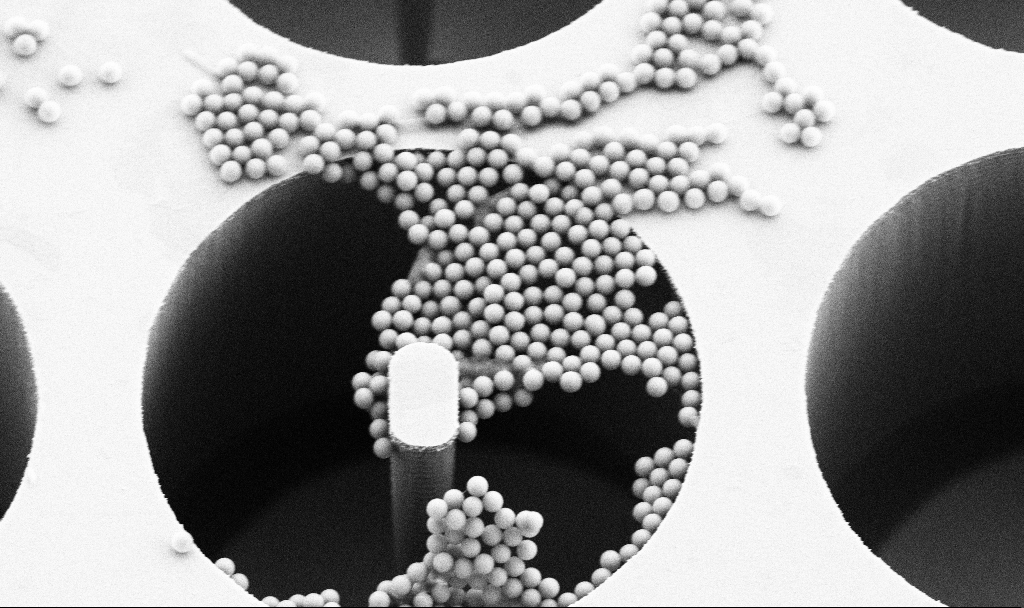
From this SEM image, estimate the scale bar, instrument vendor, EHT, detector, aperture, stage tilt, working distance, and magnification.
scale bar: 2000 nm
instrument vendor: Zeiss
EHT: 5 kV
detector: SE2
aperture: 30 µm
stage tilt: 30°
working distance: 7.1 mm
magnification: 10.58 K X